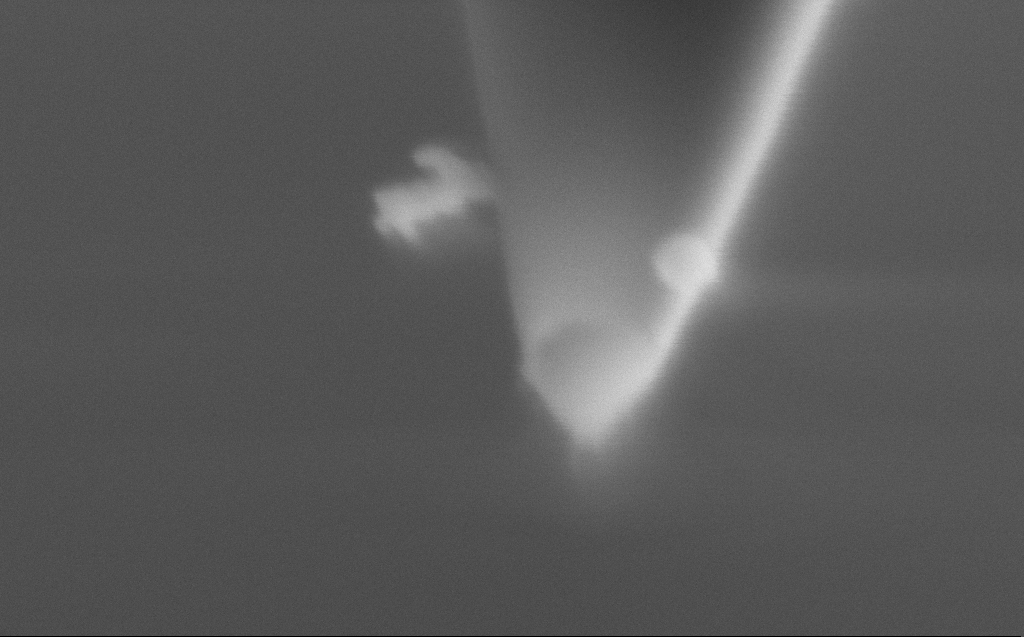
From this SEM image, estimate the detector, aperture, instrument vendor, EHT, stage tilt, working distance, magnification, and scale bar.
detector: SE2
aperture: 30 µm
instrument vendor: Zeiss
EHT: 2 kV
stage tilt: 45.1°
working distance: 5 mm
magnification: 392.07 K X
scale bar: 100 nm